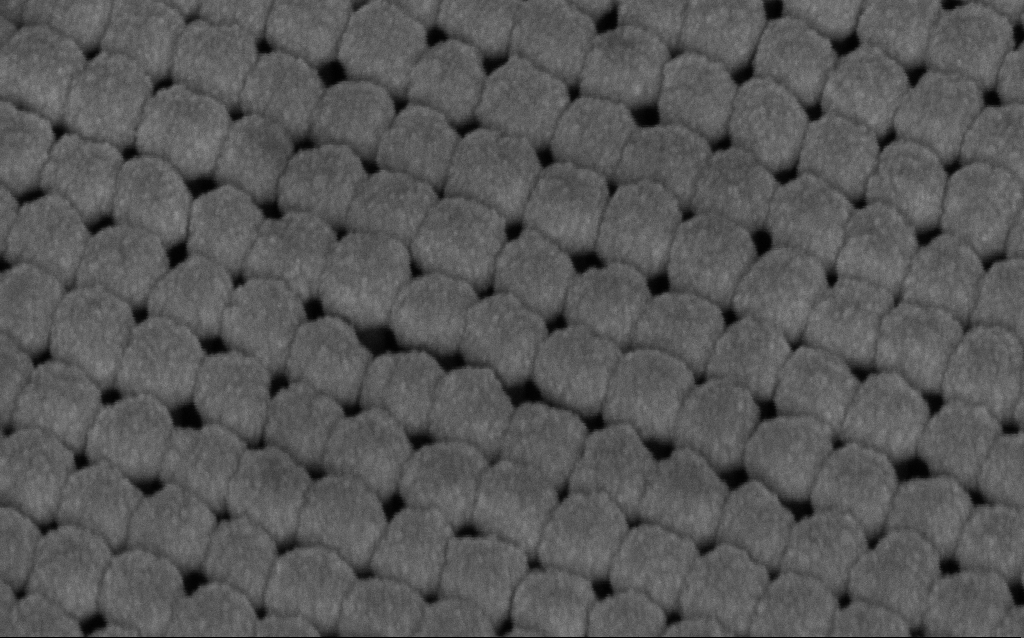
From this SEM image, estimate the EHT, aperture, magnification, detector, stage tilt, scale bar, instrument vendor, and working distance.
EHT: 5 kV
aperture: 30 µm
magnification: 170.57 K X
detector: InLens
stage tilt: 45°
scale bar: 200 nm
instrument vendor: Zeiss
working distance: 9.1 mm